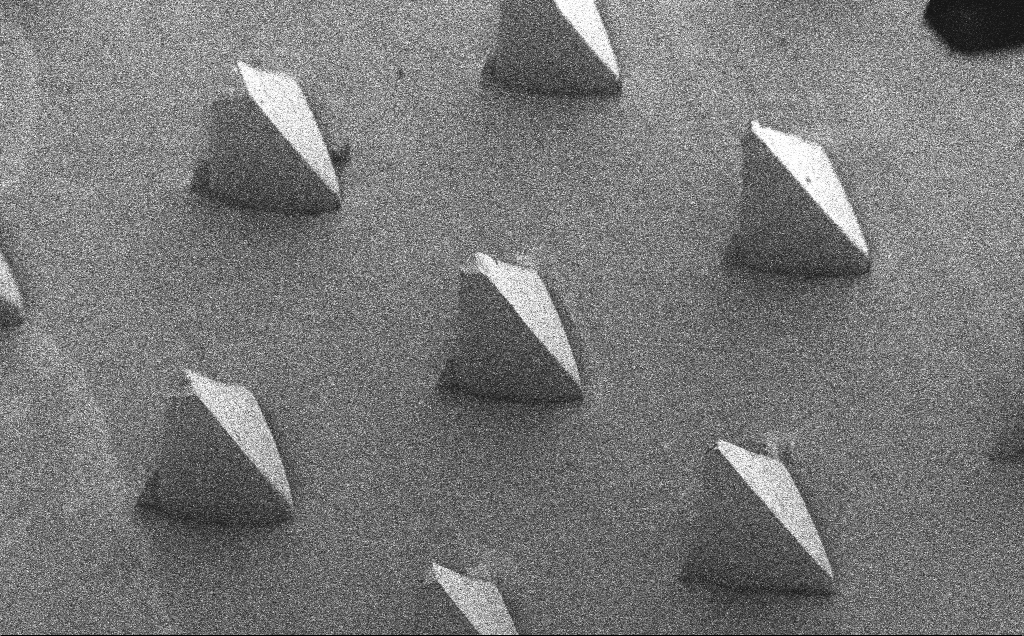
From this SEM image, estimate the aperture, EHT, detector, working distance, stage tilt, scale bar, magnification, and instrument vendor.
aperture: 30 µm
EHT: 5 kV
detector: SE2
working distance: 8 mm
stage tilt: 40°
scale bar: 200000 nm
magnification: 0.077 K X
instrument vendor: Zeiss